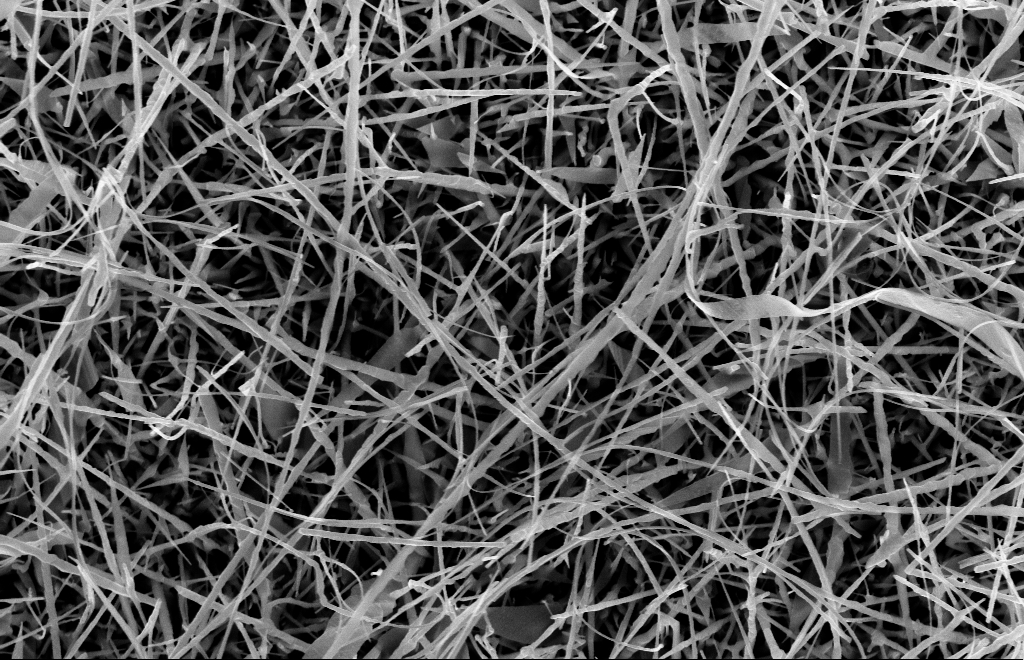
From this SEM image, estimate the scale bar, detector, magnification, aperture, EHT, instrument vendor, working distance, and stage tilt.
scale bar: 1000 nm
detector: InLens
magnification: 20 K X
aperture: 30 µm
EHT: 10 kV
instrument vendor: Zeiss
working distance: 14 mm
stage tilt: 0°